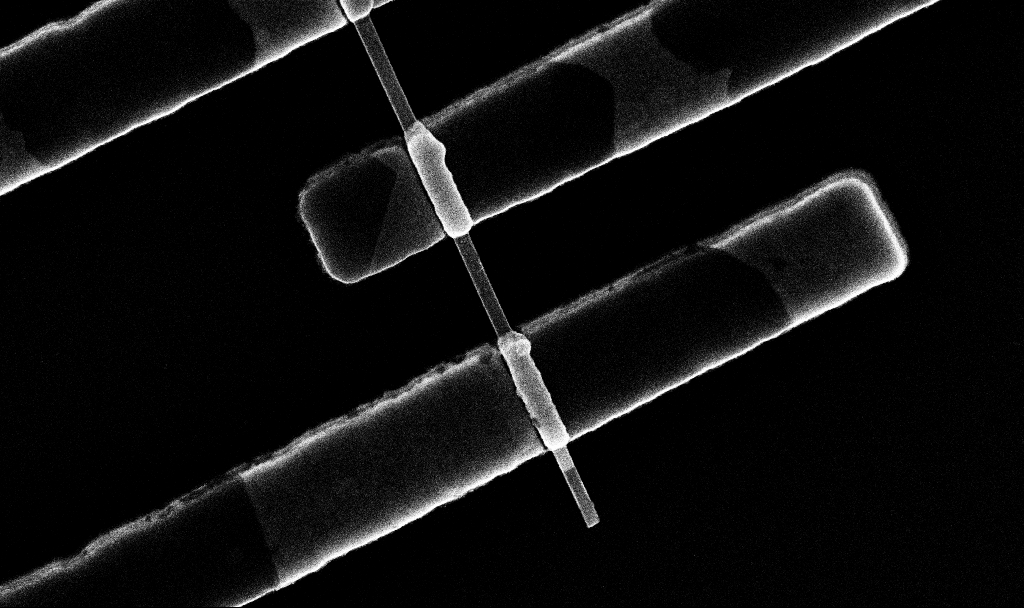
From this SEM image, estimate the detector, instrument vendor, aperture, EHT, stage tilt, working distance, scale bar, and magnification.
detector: InLens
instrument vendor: Zeiss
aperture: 30 µm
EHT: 10 kV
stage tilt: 0°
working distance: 6.8 mm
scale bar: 200 nm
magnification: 75.88 K X